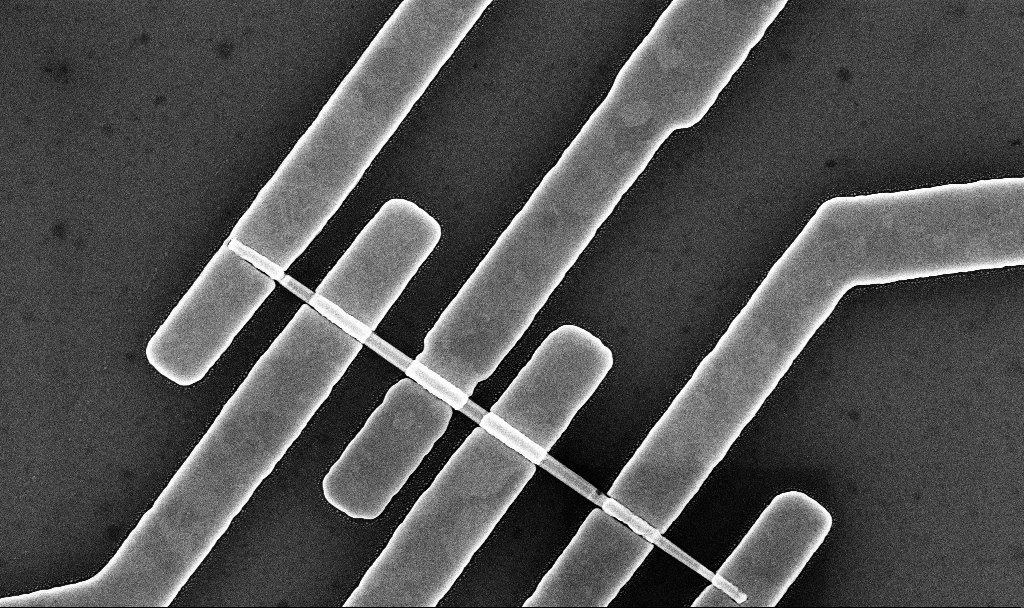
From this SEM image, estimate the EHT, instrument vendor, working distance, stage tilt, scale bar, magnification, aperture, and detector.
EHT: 10 kV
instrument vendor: Zeiss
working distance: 7.7 mm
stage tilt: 0°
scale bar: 1000 nm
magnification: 38.24 K X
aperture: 30 µm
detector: InLens